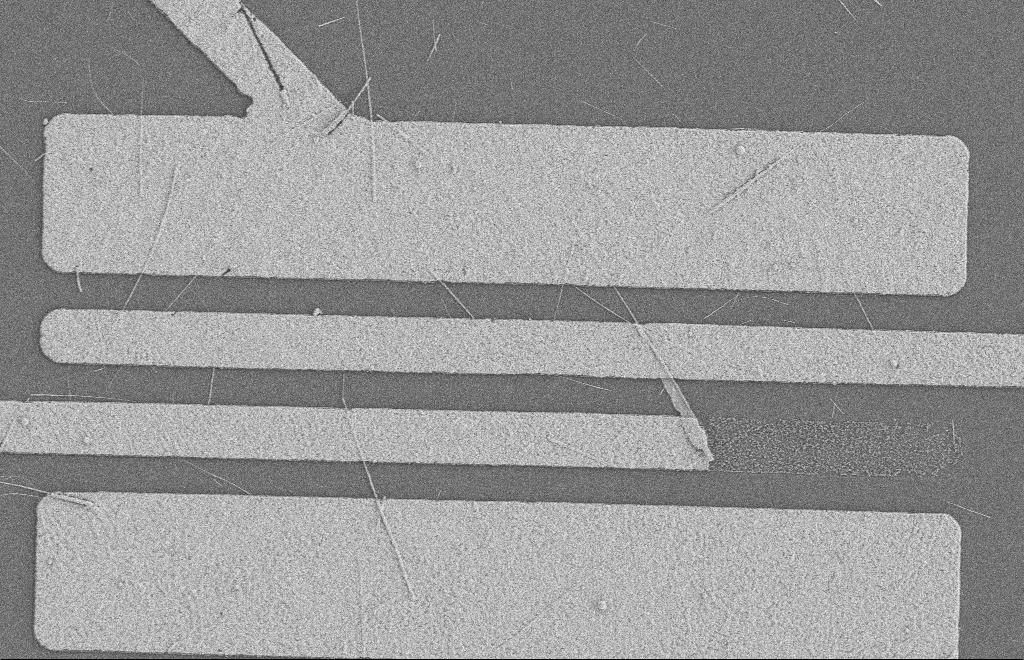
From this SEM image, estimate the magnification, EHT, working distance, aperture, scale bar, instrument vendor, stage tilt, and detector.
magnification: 5.56 K X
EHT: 2 kV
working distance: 8 mm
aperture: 20 µm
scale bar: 2000 nm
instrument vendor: Zeiss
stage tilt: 0°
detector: SE2